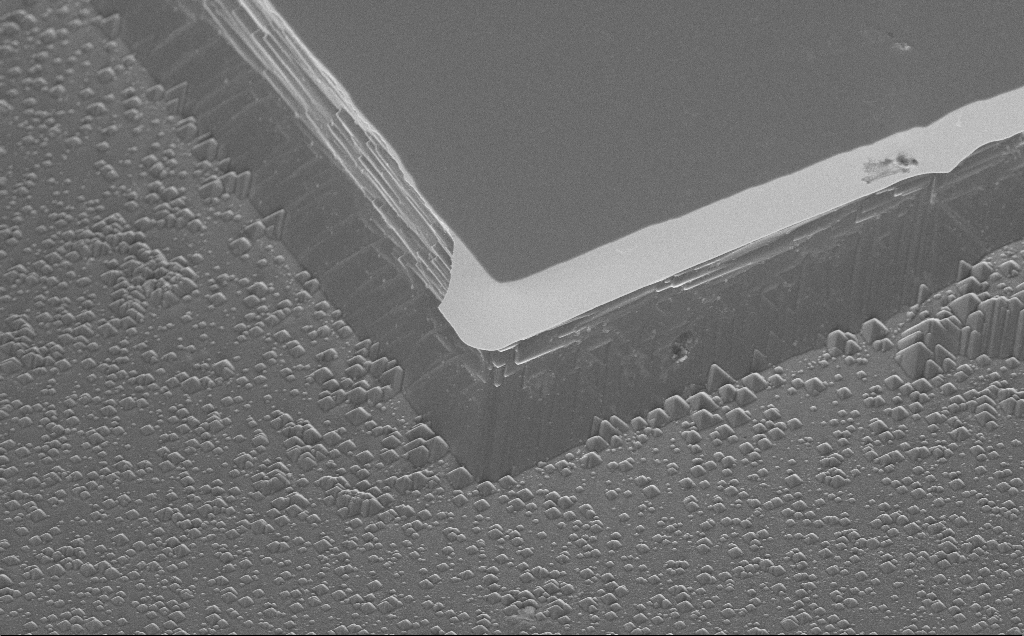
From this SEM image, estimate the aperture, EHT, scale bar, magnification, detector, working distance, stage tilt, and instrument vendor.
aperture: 30 µm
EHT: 5 kV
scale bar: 10000 nm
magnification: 4.3 K X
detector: InLens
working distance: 13 mm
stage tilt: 45°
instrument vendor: Zeiss